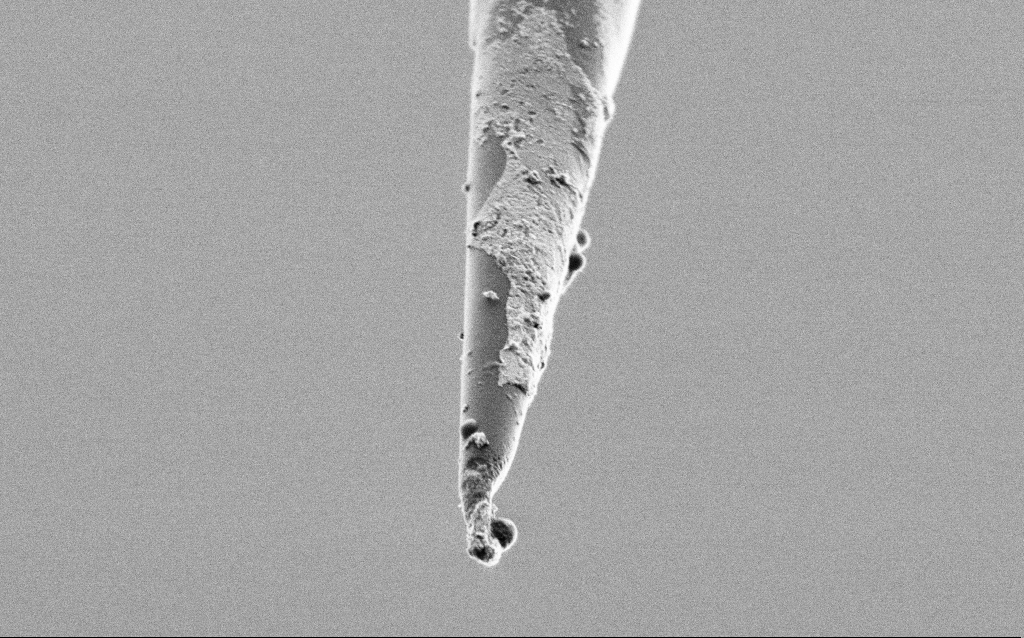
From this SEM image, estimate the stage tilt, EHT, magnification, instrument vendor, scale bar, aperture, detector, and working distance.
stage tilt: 45°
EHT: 1 kV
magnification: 25 K X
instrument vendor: Zeiss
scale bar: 2000 nm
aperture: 30 µm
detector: SE2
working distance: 6.8 mm